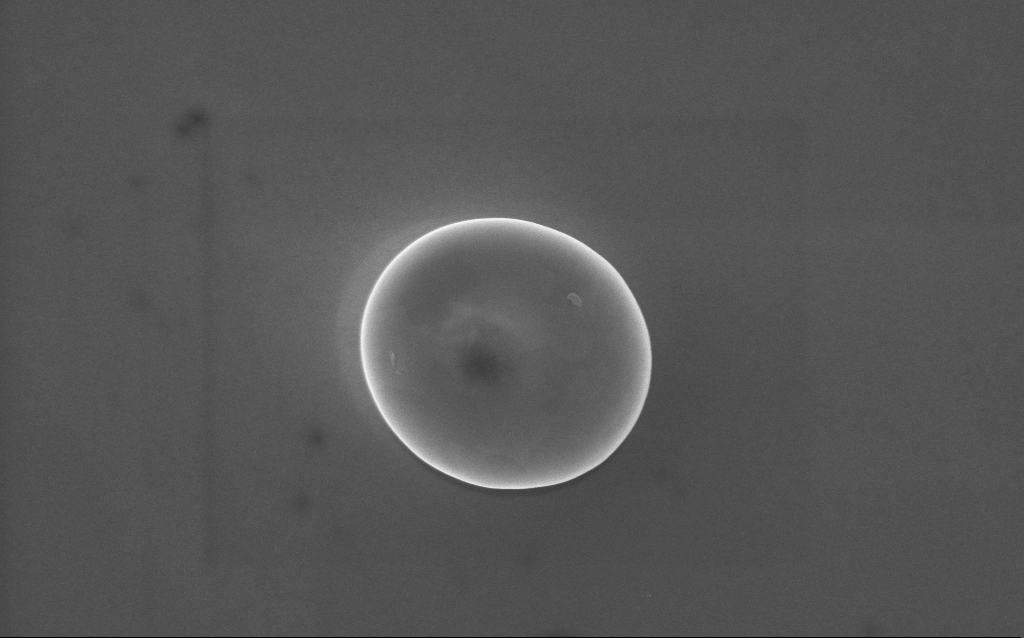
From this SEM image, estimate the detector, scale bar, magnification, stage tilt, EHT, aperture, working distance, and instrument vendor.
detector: InLens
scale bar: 1000 nm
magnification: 62 K X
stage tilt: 0°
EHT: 5 kV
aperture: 30 µm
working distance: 3 mm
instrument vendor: Zeiss